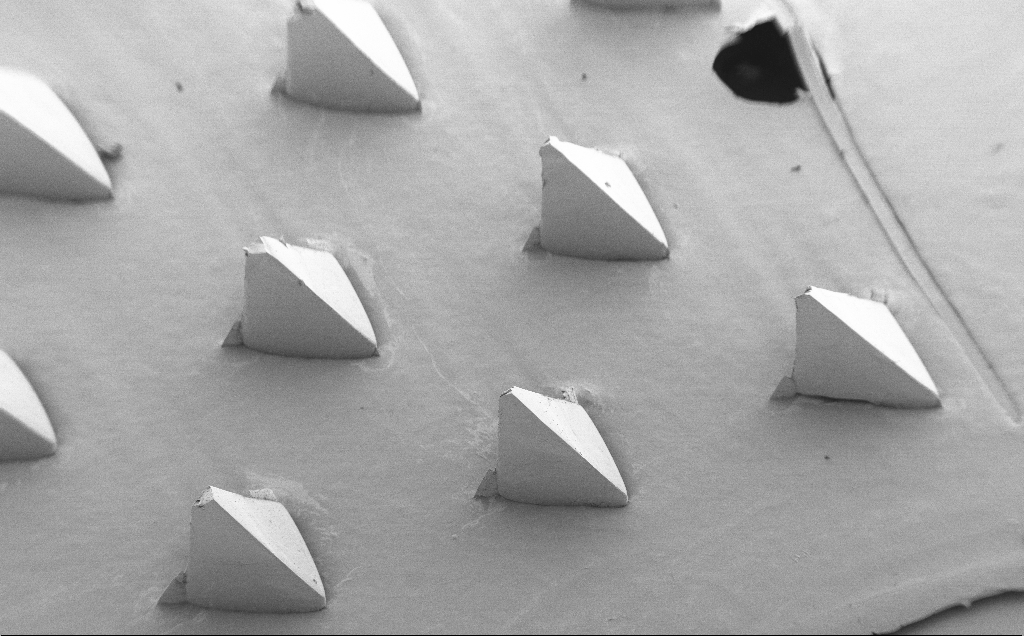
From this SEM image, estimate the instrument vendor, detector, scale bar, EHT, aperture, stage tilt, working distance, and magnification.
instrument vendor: Zeiss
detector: SE2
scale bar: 200000 nm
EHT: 5 kV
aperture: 30 µm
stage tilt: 40°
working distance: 8 mm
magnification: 0.078 K X